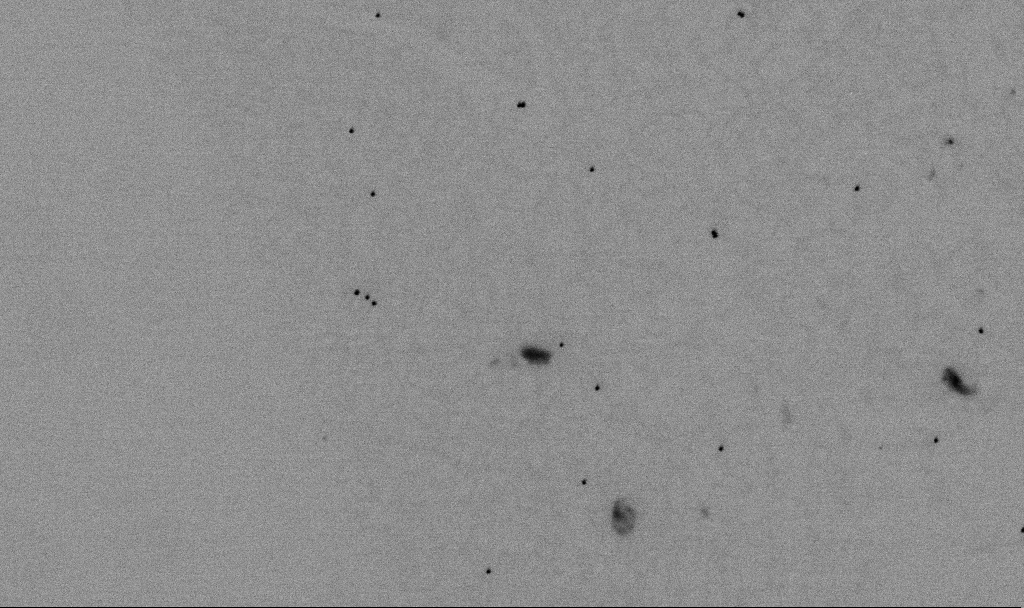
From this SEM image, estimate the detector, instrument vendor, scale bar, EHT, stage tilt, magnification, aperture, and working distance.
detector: SE2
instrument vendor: Zeiss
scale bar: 1000 nm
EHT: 2 kV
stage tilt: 0°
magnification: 60 K X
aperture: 30 µm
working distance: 5.5 mm